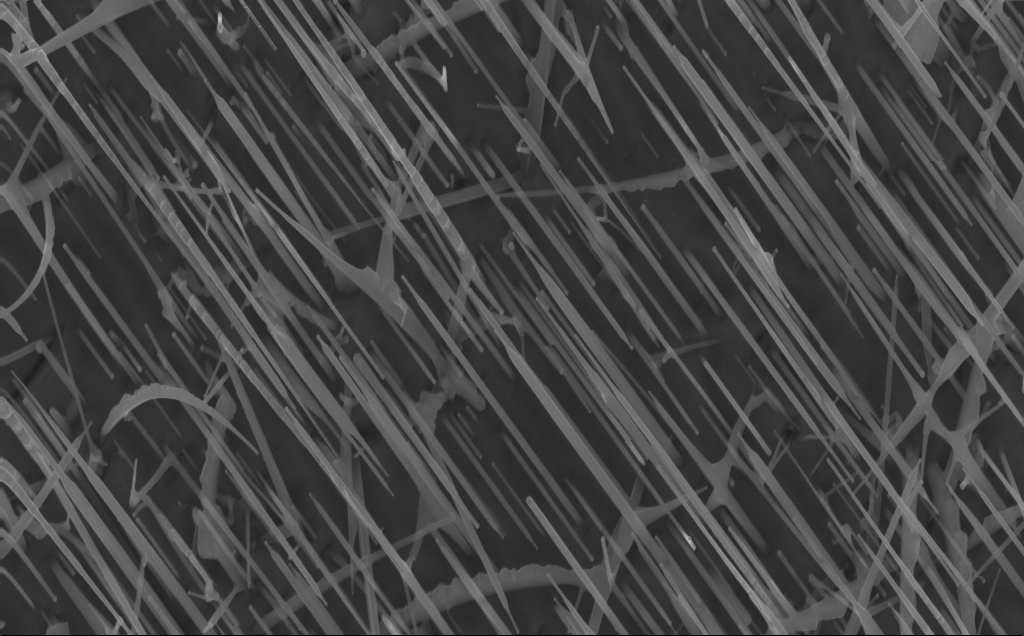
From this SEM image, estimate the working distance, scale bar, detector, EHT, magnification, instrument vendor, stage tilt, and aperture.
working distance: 4 mm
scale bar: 1000 nm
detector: InLens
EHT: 10 kV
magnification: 40 K X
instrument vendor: Zeiss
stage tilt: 0°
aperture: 30 µm